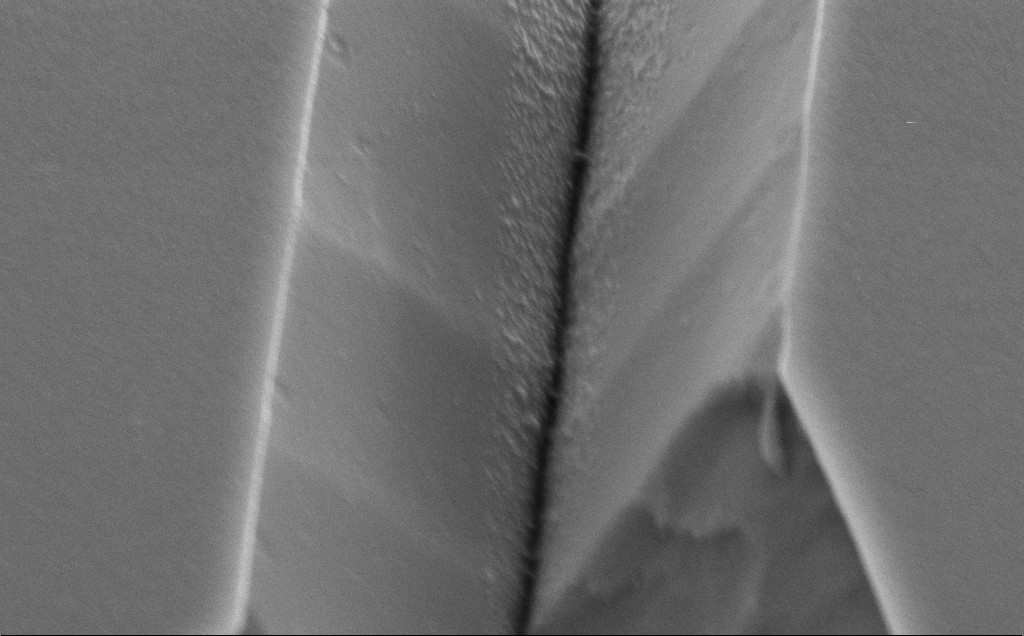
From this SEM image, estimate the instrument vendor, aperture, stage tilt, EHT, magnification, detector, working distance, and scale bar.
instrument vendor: Zeiss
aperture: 30 µm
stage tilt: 50°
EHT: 5 kV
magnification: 62 K X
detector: SE2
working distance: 10 mm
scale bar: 1000 nm